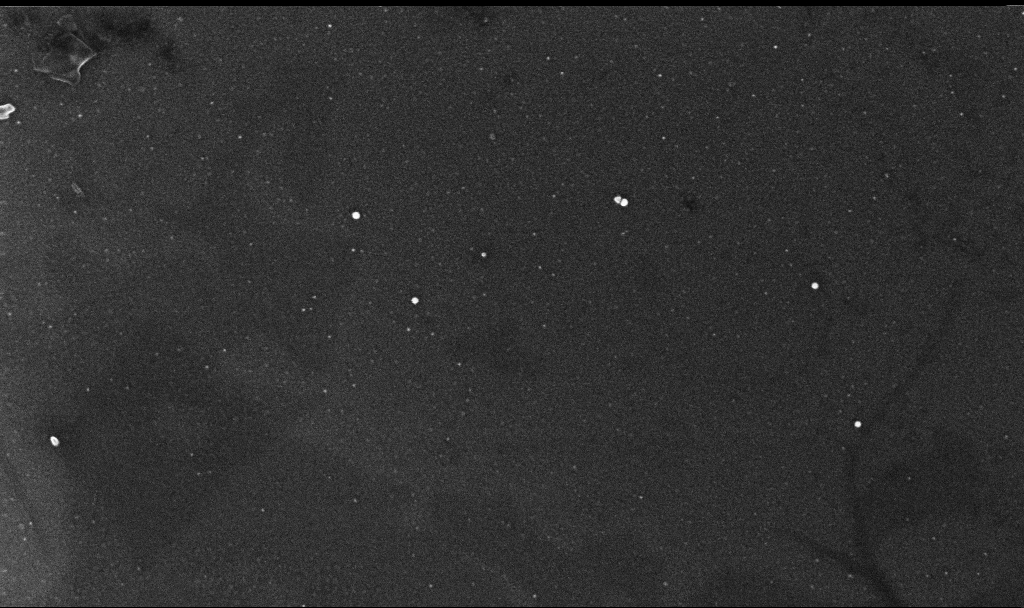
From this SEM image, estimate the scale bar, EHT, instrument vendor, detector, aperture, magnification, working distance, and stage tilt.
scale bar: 200 nm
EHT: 10 kV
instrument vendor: Zeiss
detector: InLens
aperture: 30 µm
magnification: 101.12 K X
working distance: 3 mm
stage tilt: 0°